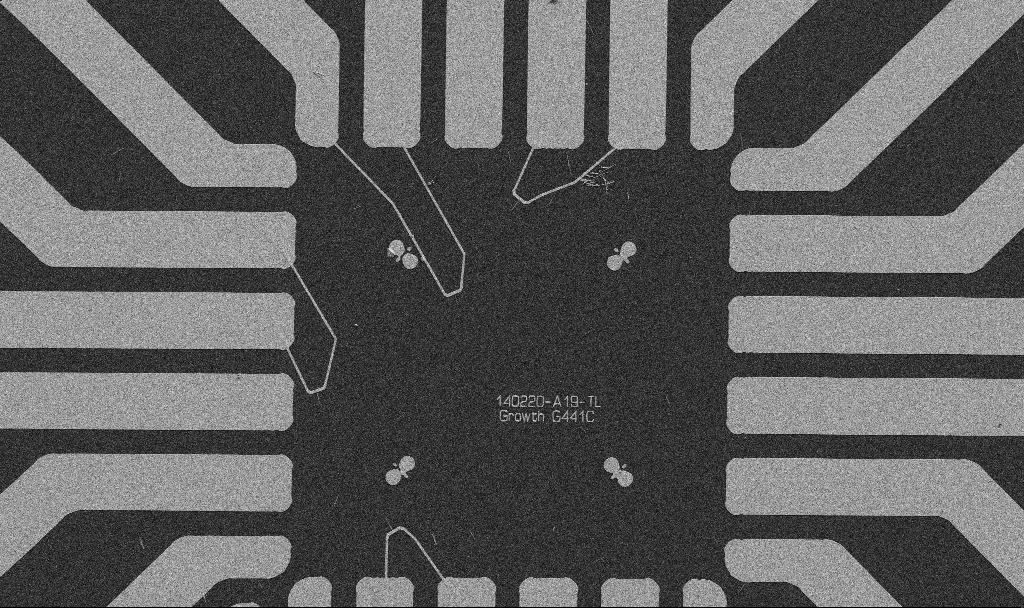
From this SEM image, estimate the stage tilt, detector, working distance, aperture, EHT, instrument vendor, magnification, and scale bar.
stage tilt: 0°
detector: SE2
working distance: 10.7 mm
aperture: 30 µm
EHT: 5 kV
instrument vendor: Zeiss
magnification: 1 K X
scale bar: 20000 nm